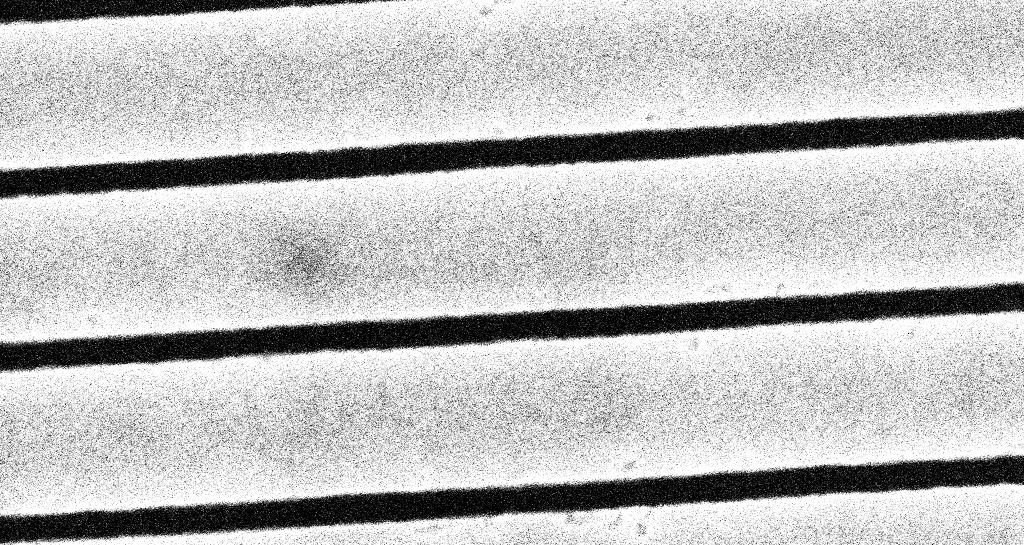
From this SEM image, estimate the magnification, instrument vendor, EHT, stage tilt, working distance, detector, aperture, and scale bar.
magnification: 129.79 K X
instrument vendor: Zeiss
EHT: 10 kV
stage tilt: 0°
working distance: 2 mm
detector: InLens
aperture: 30 µm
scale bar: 200 nm